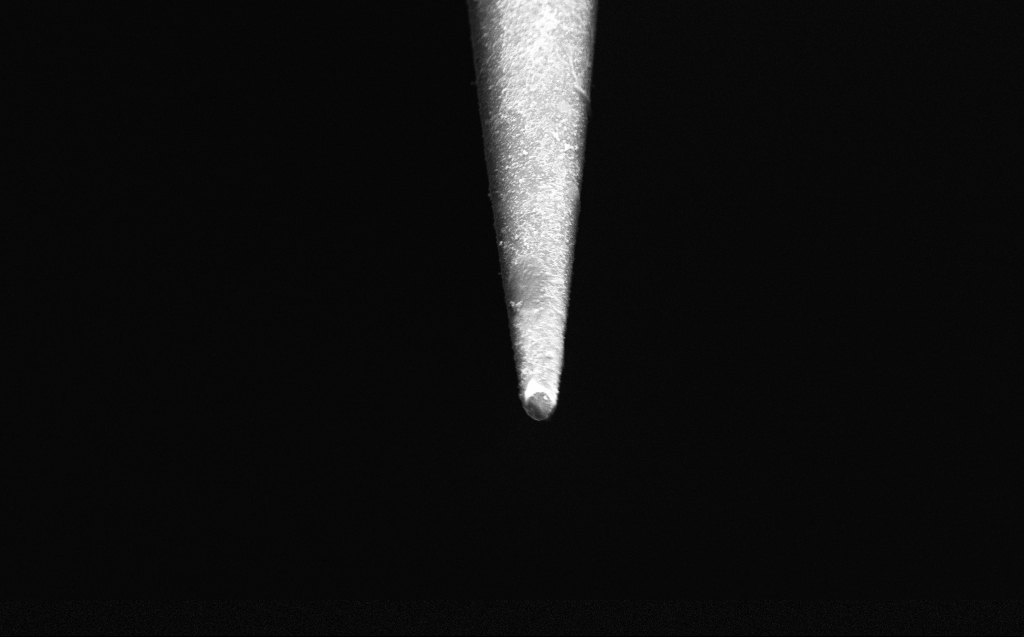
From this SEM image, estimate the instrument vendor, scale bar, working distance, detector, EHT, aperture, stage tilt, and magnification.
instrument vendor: Zeiss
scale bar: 2000 nm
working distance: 4 mm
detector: InLens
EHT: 2 kV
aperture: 30 µm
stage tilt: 45°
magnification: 25 K X